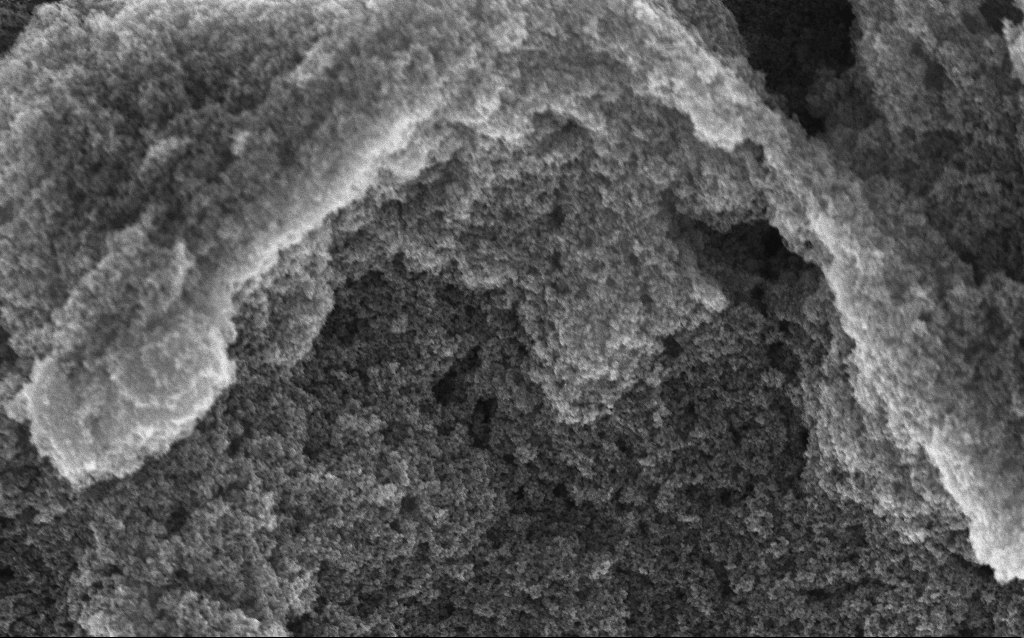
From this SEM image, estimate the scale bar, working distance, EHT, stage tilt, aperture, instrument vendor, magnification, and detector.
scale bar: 1000 nm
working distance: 2.8 mm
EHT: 10 kV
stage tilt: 0°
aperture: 30 µm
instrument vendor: Zeiss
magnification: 37.88 K X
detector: InLens